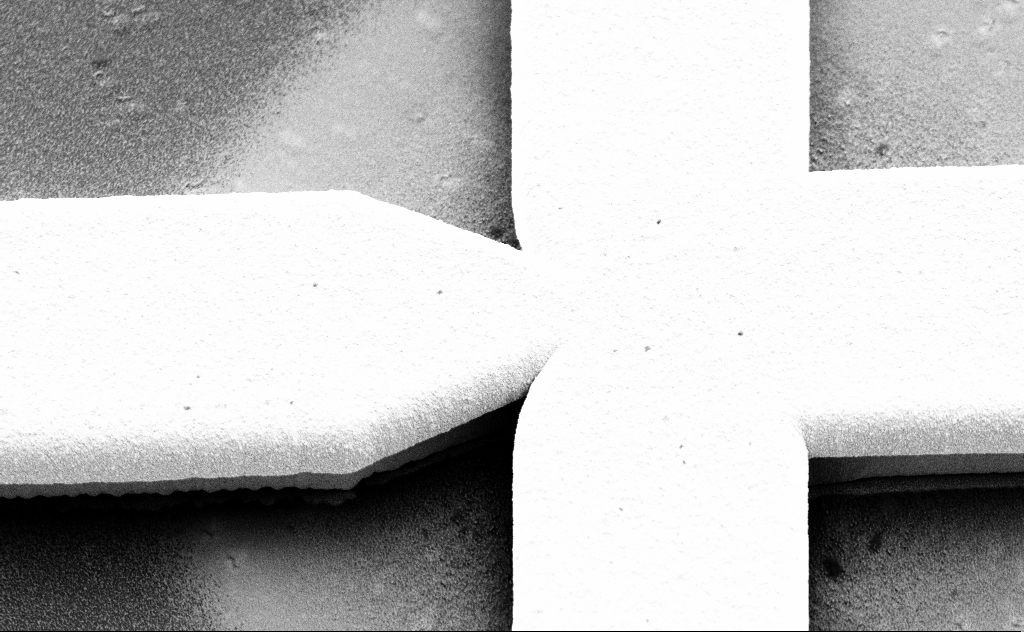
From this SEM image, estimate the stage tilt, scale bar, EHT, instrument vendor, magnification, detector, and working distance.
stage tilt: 45°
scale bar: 2000 nm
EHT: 3 kV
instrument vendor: Zeiss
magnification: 8.66 K X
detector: SE2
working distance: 12 mm